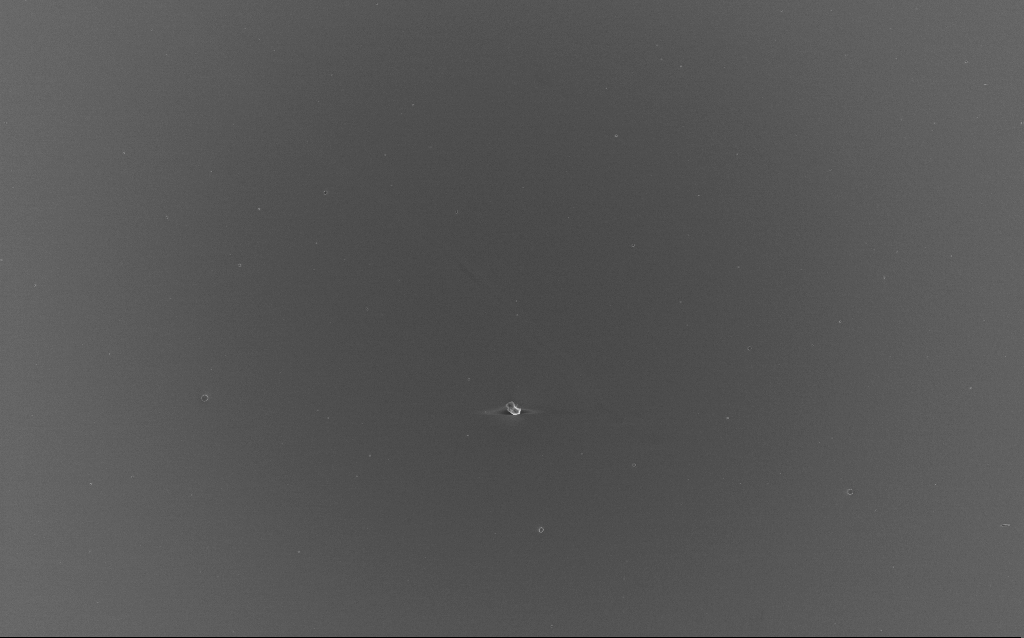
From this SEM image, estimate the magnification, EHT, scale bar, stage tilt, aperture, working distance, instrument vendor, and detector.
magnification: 0.623 K X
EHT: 10 kV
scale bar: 100000 nm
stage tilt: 0°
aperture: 30 µm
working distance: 4 mm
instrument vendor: Zeiss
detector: InLens